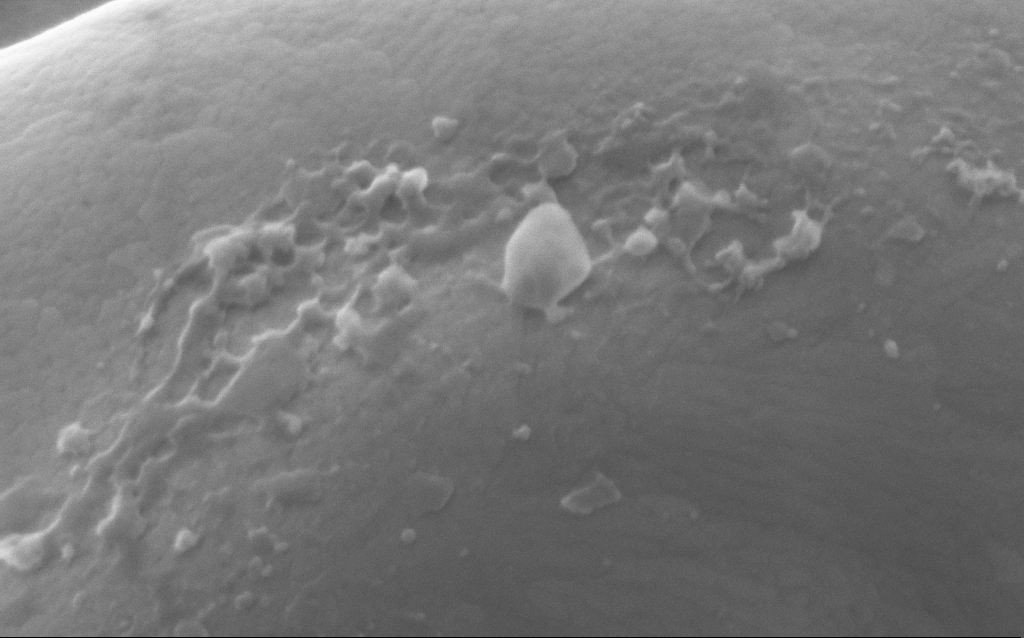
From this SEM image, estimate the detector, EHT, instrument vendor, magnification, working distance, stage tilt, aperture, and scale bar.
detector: InLens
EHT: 5 kV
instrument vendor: Zeiss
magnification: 254.03 K X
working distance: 3 mm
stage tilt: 0°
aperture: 30 µm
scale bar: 100 nm